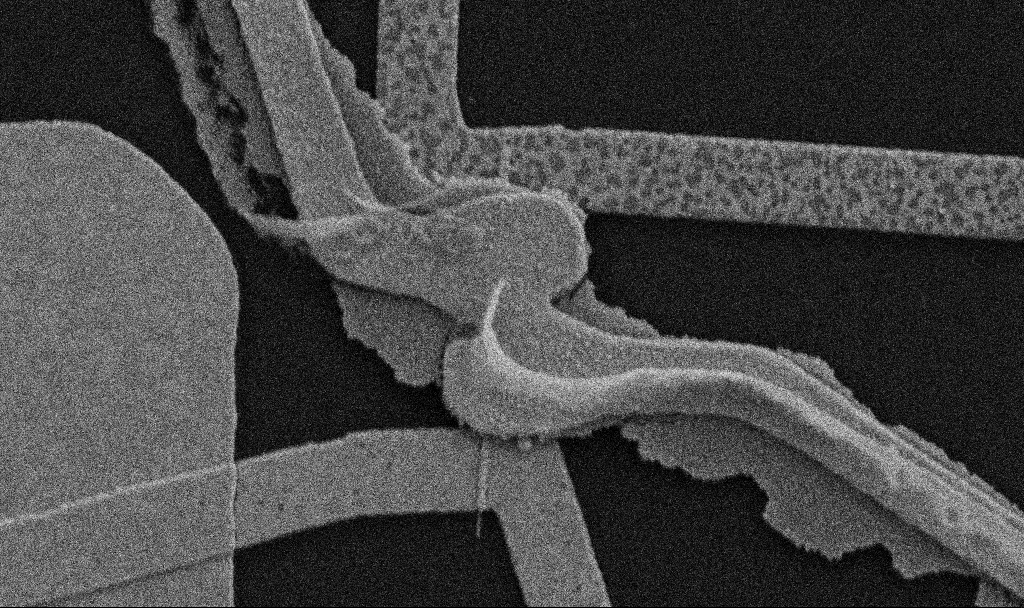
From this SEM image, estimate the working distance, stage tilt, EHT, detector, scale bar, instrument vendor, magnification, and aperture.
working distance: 10.7 mm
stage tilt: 0°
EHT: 5 kV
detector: SE2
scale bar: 1000 nm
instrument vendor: Zeiss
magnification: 30 K X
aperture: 30 µm